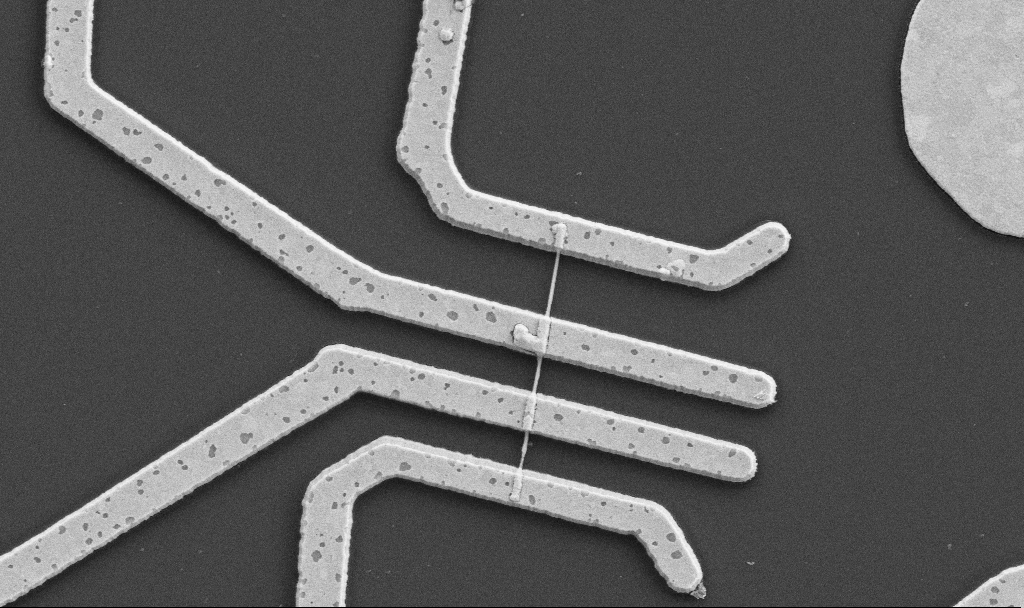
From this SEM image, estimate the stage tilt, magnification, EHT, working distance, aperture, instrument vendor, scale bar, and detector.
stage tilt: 0°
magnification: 20 K X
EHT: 5 kV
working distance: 10.7 mm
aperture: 30 µm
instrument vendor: Zeiss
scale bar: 1000 nm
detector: SE2